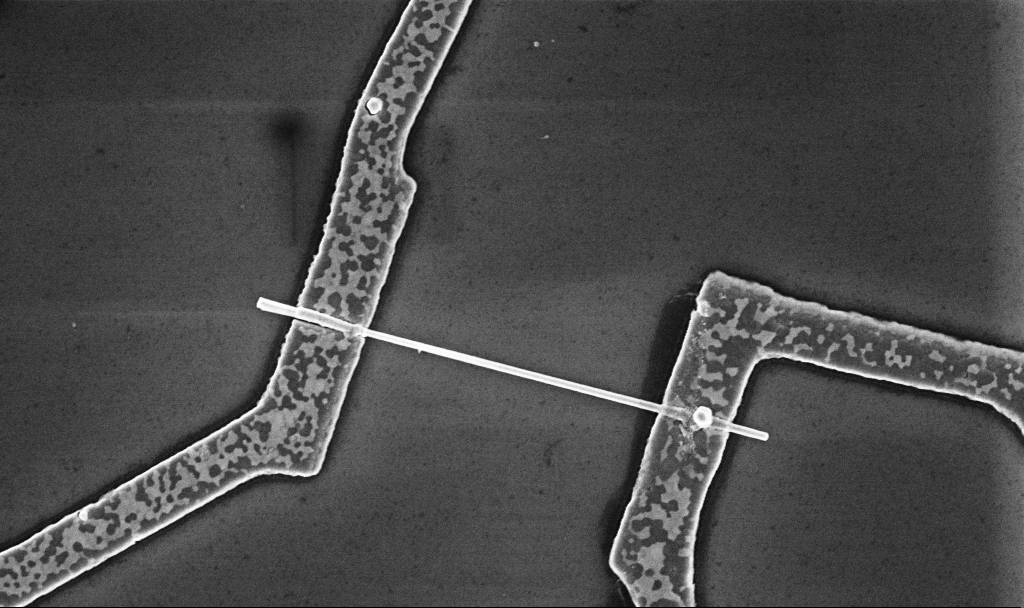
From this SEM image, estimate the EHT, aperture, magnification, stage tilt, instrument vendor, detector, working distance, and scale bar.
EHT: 5 kV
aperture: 30 µm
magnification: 30 K X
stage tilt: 0°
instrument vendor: Zeiss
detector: InLens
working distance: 8.7 mm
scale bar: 1000 nm